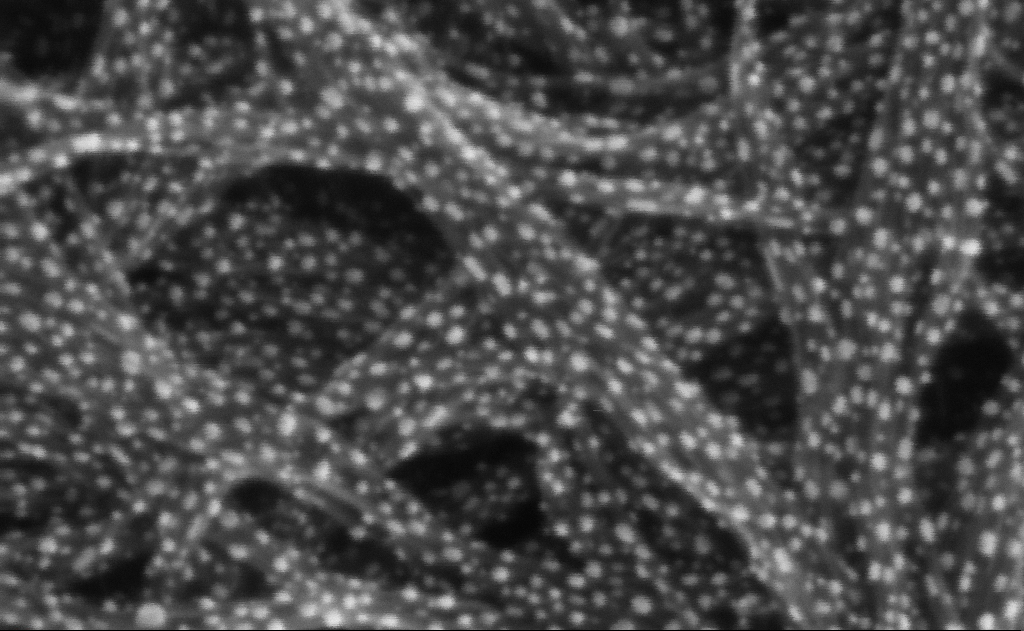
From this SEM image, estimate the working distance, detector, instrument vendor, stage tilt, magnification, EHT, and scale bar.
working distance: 3 mm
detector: InLens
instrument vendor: Zeiss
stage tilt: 0°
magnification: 1026.87 K X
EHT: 10 kV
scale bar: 20 nm